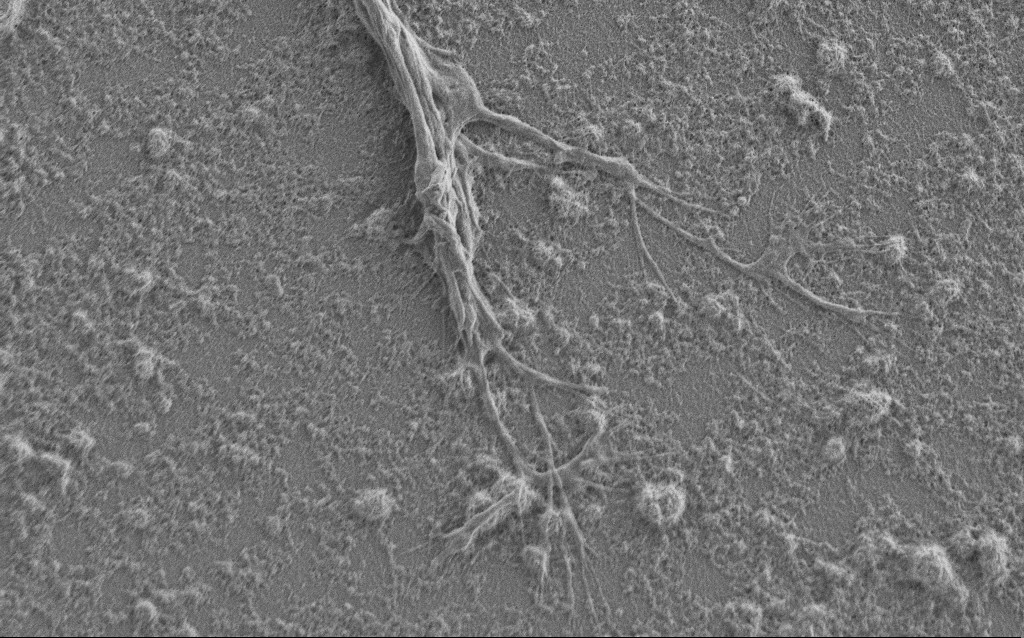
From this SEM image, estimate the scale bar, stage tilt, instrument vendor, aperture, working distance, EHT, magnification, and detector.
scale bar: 2000 nm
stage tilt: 0°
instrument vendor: Zeiss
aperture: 30 µm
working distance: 6 mm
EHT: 1 kV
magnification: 7.5 K X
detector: SE2